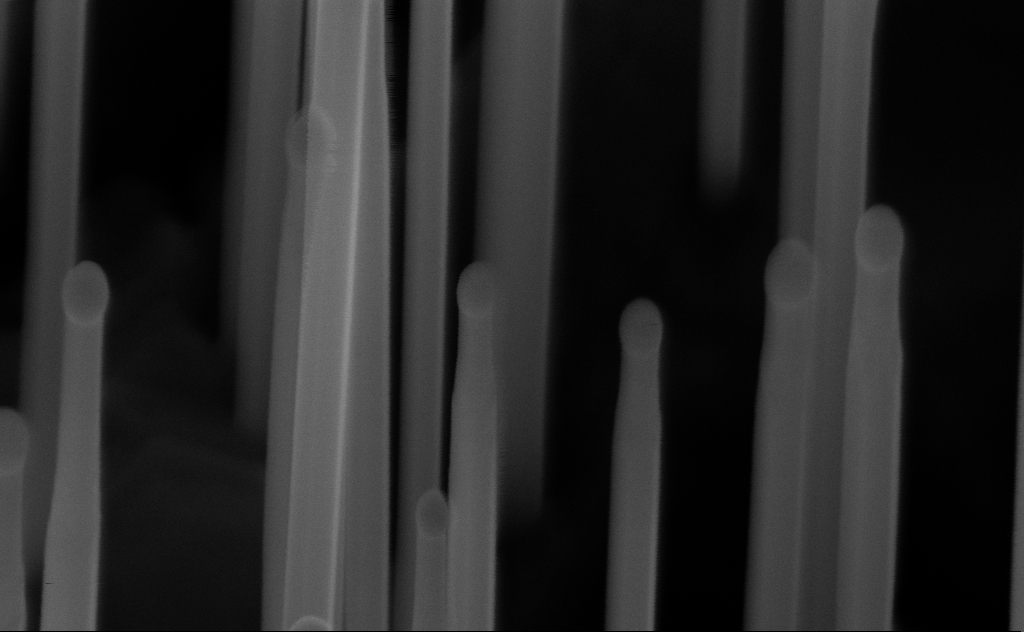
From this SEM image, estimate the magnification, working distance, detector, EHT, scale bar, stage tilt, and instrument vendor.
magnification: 248.47 K X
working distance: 6 mm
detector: InLens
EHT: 10 kV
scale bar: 200 nm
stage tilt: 45°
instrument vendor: Zeiss